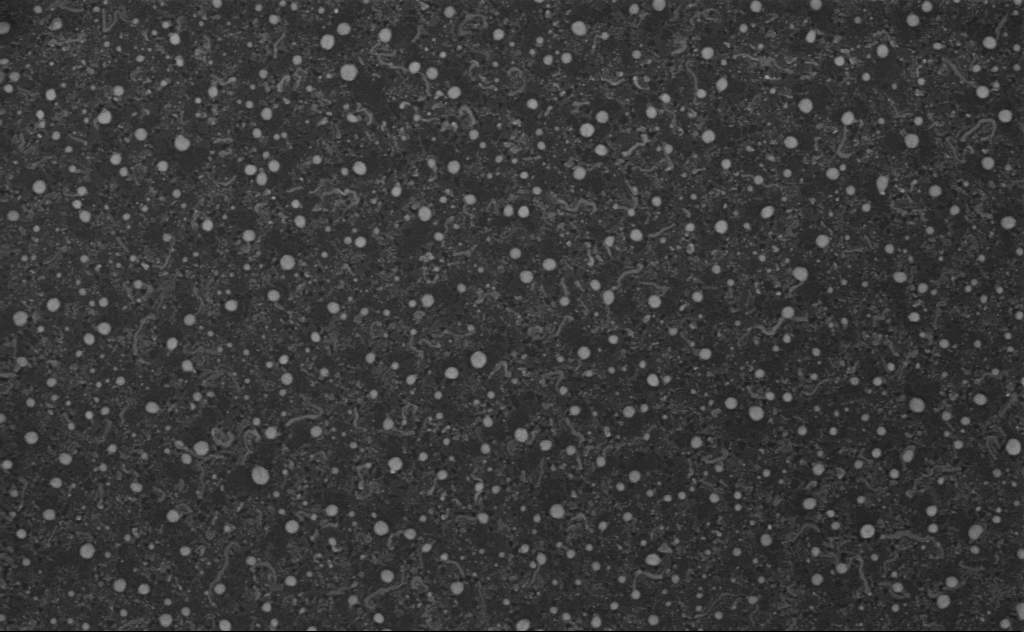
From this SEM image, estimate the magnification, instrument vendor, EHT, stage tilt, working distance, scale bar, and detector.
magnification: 80 K X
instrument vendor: Zeiss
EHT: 3 kV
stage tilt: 0°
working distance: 4 mm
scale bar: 200 nm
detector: InLens